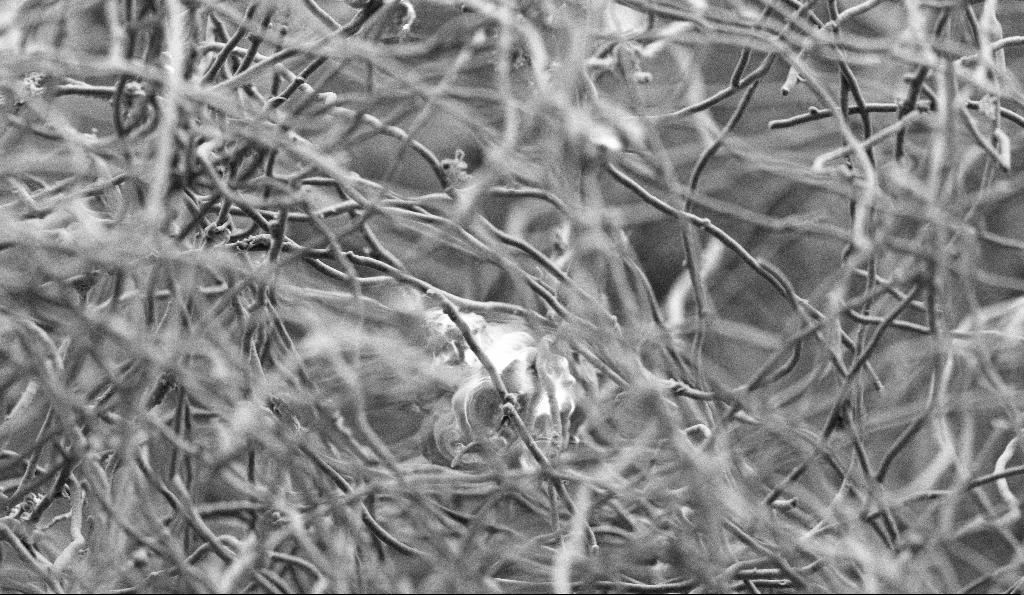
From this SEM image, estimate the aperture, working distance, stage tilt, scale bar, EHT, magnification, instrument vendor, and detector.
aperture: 30 µm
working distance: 5.1 mm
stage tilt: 0°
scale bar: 10000 nm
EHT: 3 kV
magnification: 5 K X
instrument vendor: Zeiss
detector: SE2